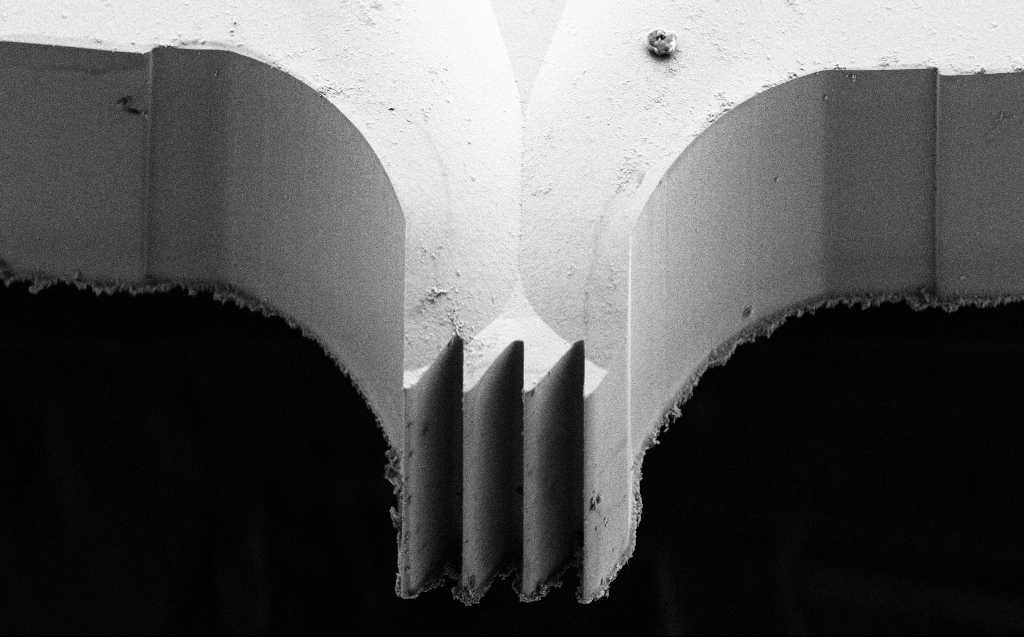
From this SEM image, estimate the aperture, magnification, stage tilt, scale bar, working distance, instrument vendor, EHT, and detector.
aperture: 30 µm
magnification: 1.98 K X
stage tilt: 45°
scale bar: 10000 nm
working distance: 5 mm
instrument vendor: Zeiss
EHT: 5 kV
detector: SE2